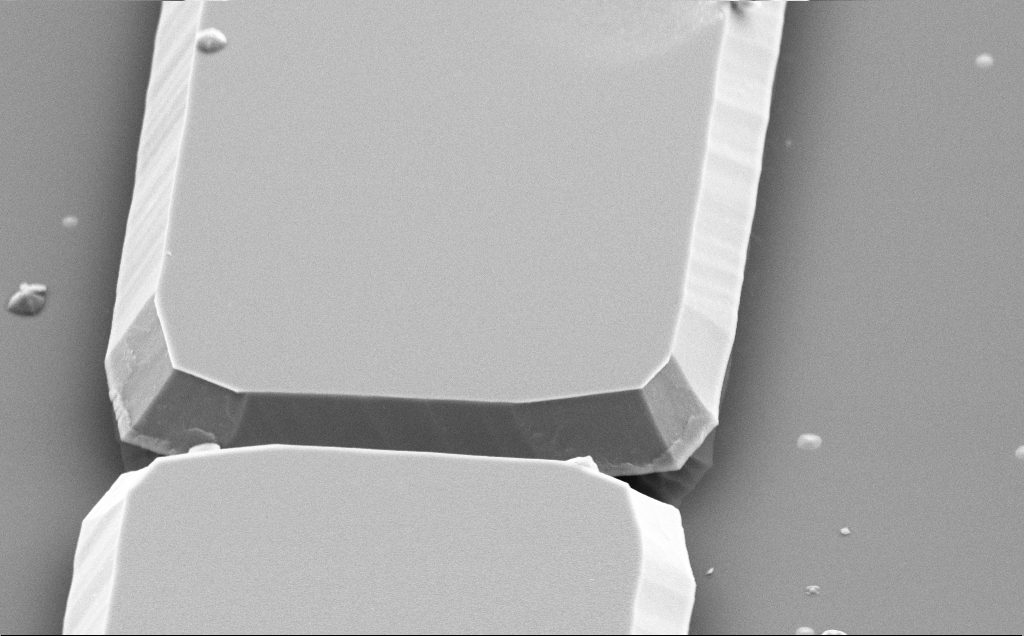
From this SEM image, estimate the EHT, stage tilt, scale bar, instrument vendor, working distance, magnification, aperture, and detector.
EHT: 5 kV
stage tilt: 50°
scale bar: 2000 nm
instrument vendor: Zeiss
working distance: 12 mm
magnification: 10.23 K X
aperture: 30 µm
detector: SE2